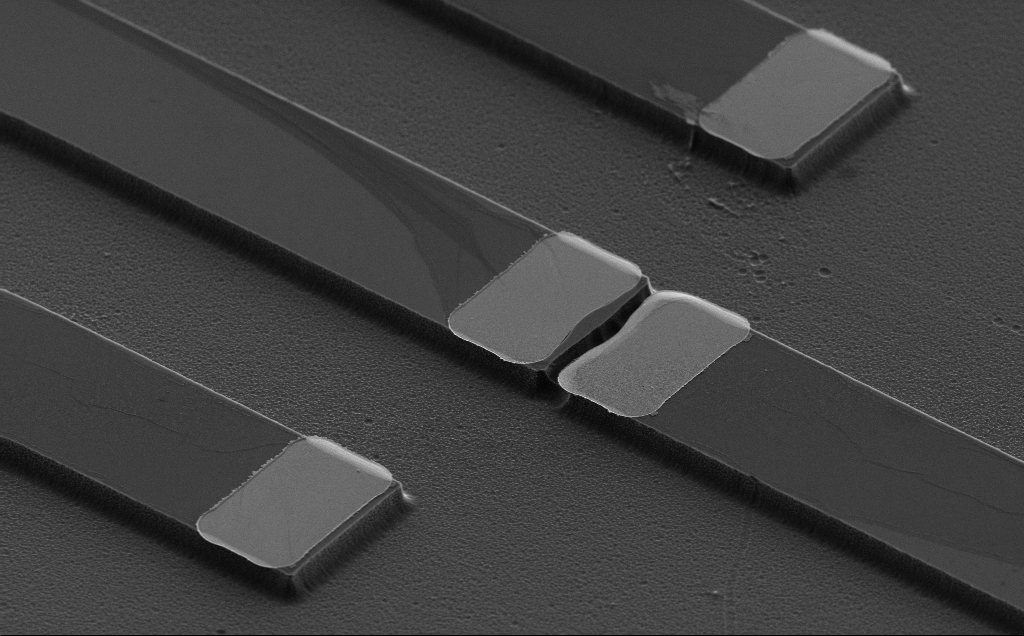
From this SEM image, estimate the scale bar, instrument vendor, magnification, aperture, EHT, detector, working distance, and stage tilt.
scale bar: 10000 nm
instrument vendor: Zeiss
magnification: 3.74 K X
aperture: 30 µm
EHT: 5 kV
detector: SE2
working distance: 10 mm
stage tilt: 50°